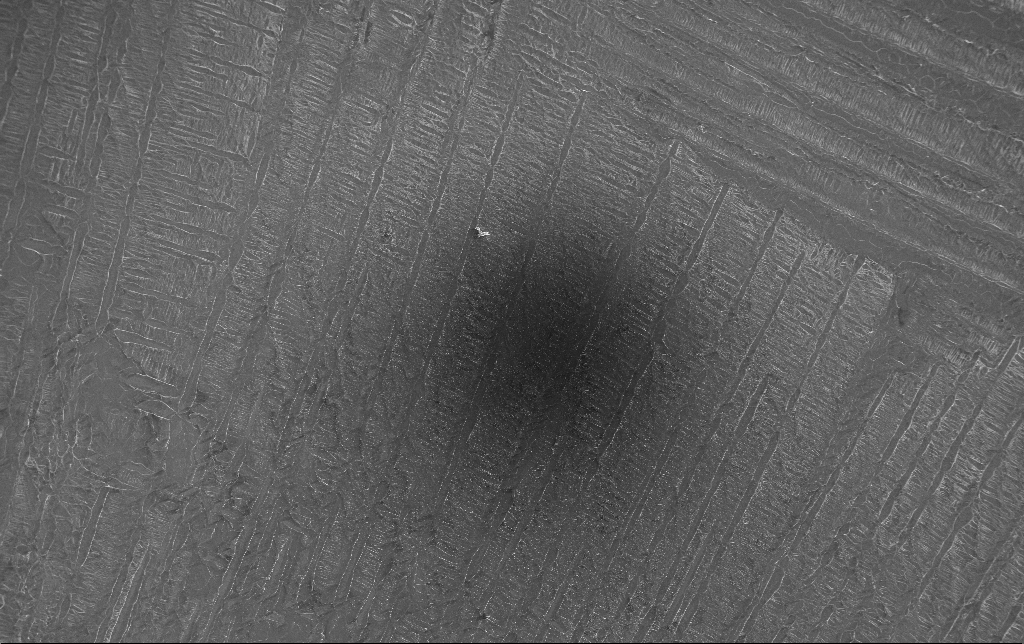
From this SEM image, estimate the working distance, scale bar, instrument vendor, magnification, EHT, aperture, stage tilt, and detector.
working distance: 3.1 mm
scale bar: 100000 nm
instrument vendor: Zeiss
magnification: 0.152 K X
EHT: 5 kV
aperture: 30 µm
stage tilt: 0°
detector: InLens